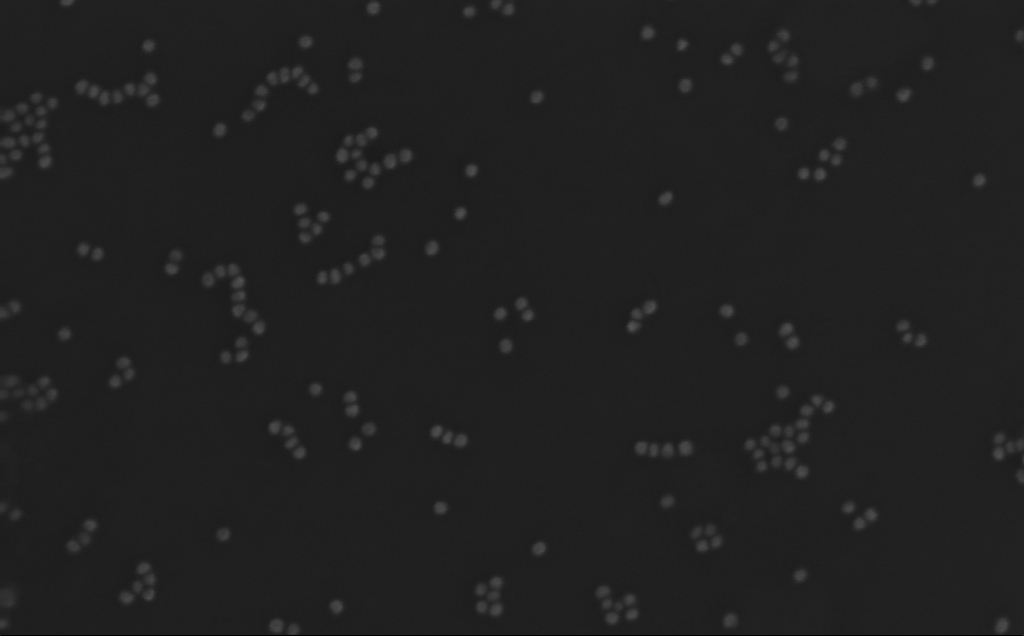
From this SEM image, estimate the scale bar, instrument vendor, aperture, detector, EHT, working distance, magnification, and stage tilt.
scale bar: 200 nm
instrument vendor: Zeiss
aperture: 30 µm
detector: InLens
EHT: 10 kV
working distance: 3 mm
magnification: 270.54 K X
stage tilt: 0°